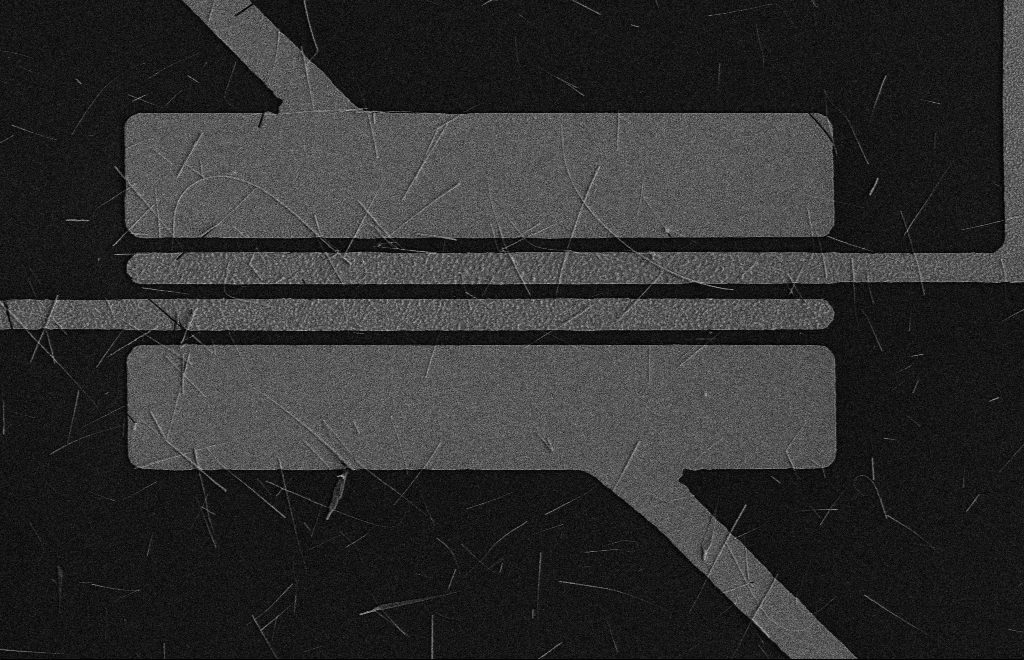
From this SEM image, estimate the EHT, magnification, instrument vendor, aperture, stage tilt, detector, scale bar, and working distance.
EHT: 5 kV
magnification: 4.3 K X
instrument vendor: Zeiss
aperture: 10 µm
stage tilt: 0°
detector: SE2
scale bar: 10000 nm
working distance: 16 mm